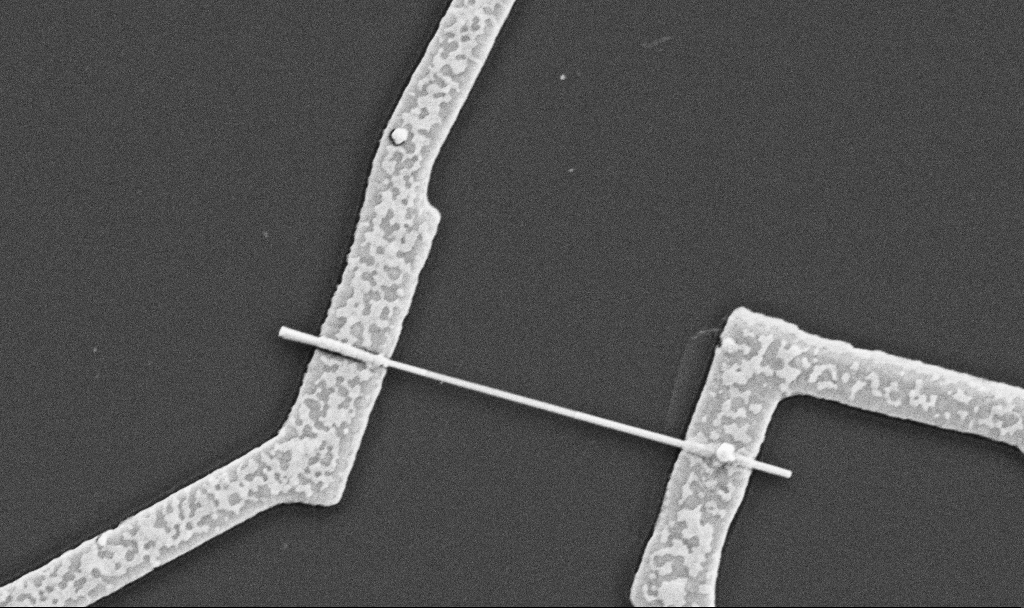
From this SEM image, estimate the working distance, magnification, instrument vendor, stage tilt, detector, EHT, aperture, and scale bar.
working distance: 8.7 mm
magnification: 30 K X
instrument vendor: Zeiss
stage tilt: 0°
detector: SE2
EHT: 5 kV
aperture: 30 µm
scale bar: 1000 nm